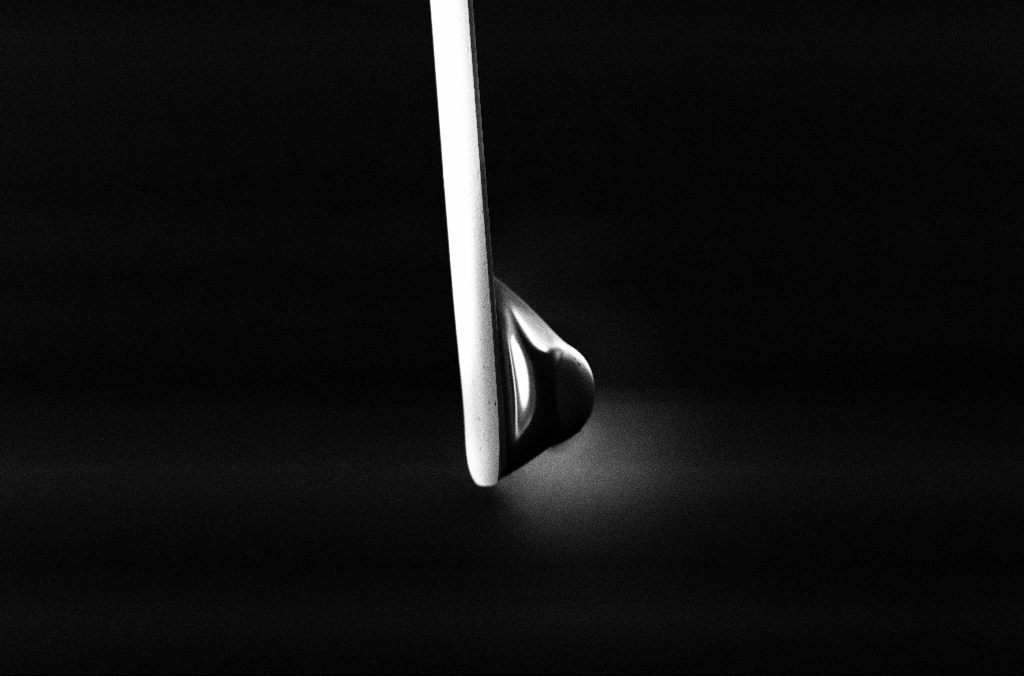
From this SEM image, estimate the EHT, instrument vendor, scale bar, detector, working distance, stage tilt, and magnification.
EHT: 5 kV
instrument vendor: Zeiss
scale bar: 10000 nm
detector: InLens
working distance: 5.3 mm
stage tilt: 0°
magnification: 2.5 K X